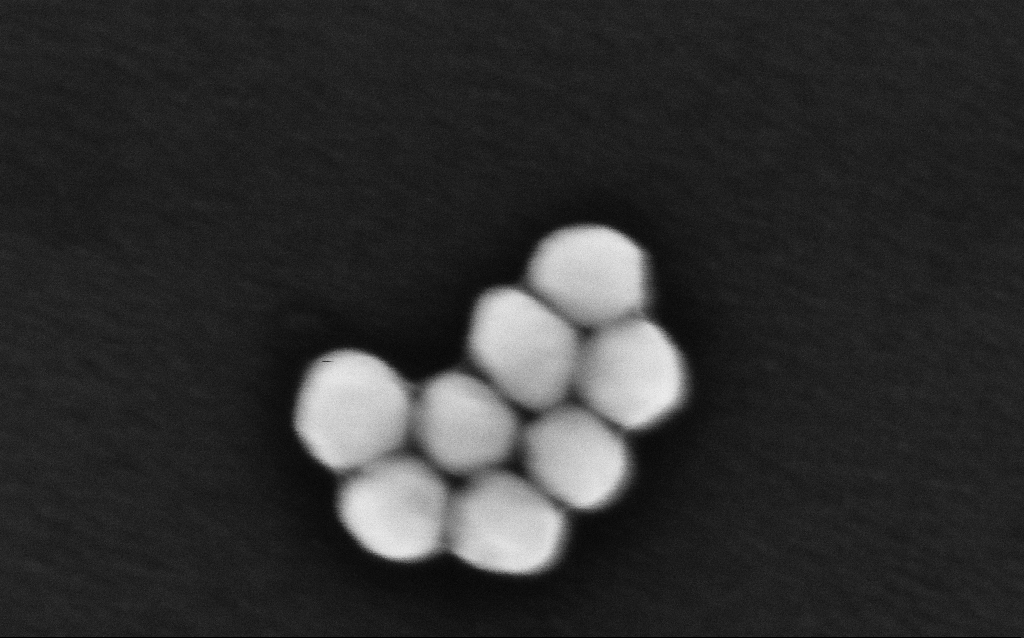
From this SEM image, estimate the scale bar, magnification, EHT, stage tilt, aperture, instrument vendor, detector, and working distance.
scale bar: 100 nm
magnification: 672.55 K X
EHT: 8 kV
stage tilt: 0°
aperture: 30 µm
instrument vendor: Zeiss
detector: InLens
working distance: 3.2 mm